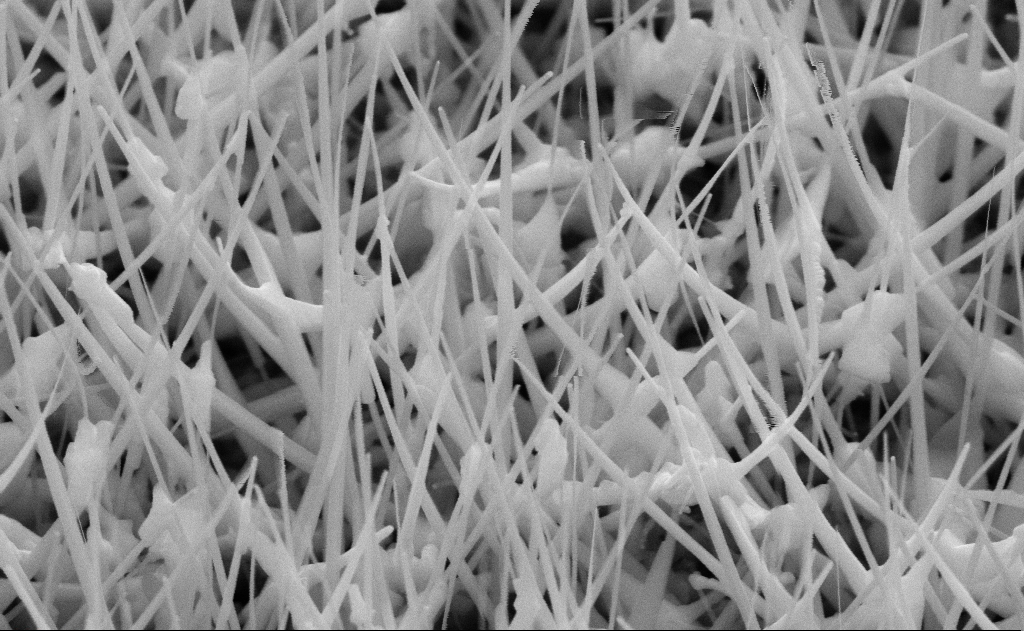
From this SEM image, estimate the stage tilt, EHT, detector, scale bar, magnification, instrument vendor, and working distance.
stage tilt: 45°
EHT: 10 kV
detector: SE2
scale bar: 200 nm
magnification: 80 K X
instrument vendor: Zeiss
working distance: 11 mm